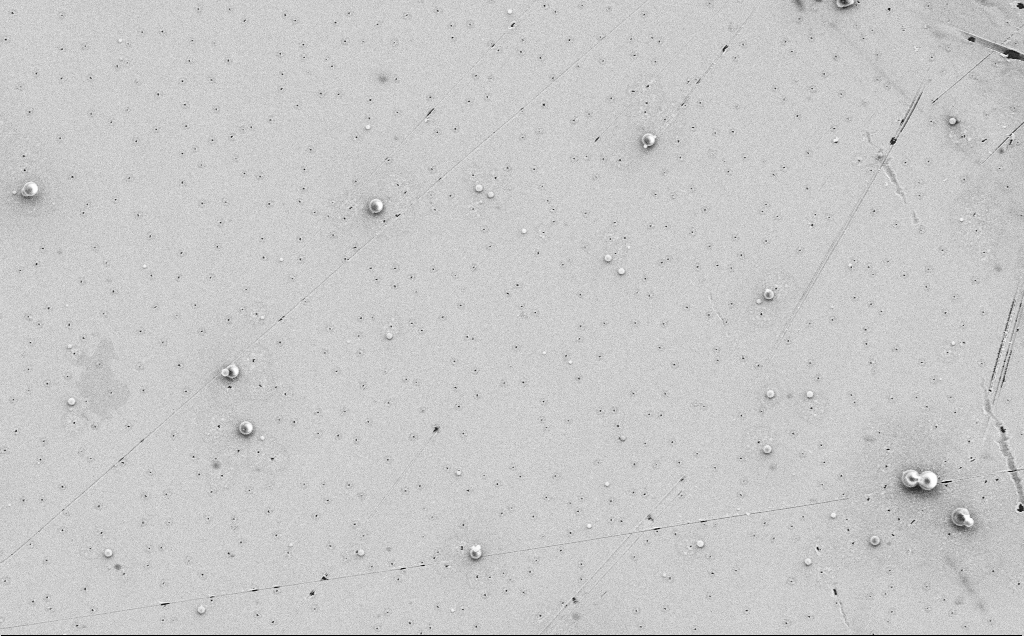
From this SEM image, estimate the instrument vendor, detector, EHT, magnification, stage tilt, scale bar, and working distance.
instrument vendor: Zeiss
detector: SE2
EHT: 5 kV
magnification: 4.2 K X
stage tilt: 0°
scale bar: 10000 nm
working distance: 12 mm